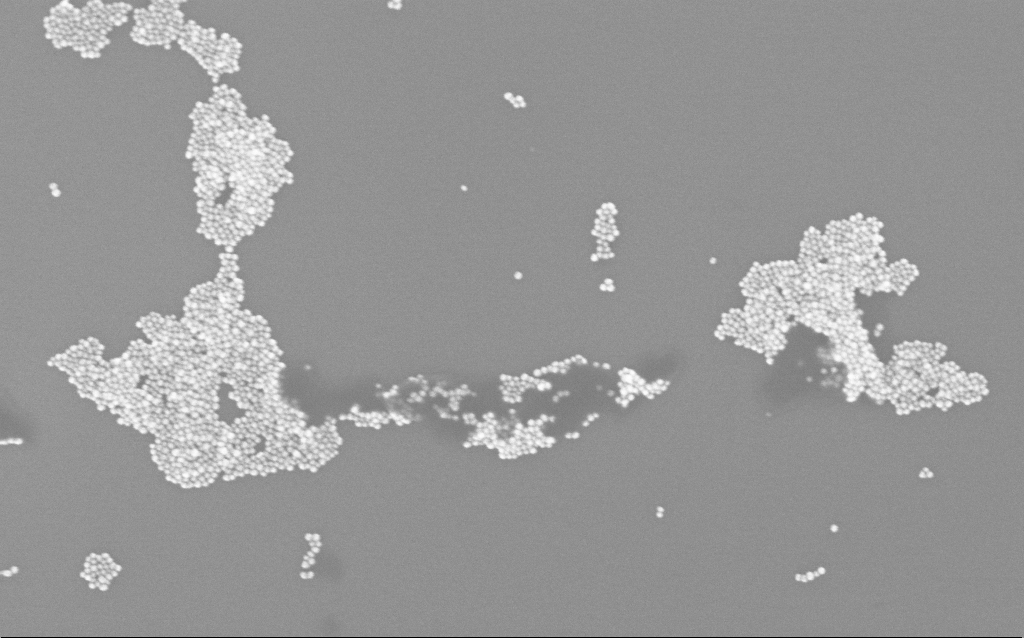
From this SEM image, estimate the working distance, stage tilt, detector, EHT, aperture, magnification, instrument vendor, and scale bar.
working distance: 3.2 mm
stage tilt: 0°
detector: InLens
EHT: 10 kV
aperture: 30 µm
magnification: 144.41 K X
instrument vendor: Zeiss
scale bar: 200 nm